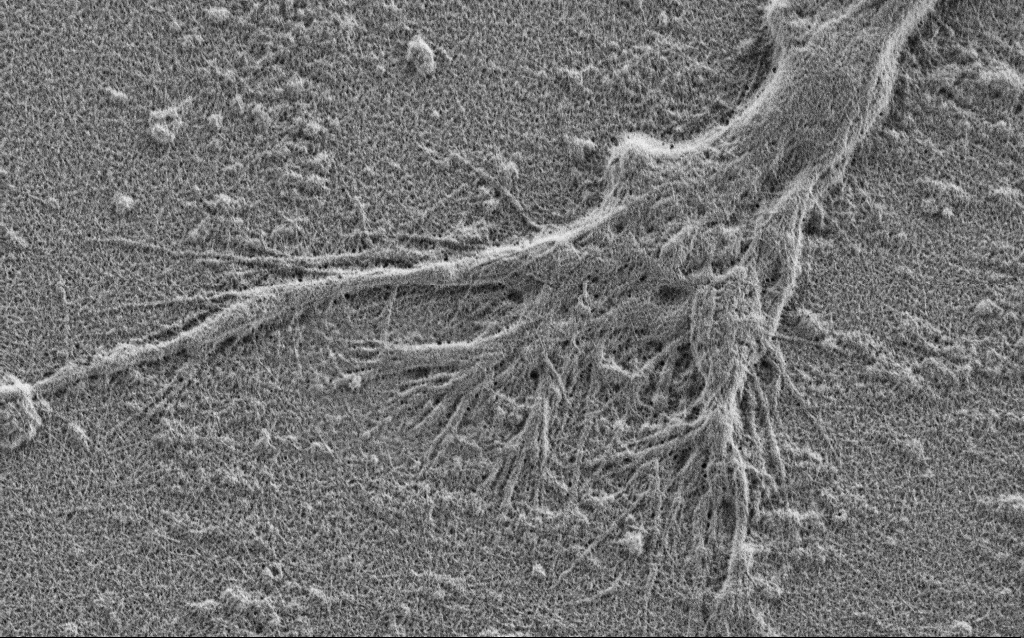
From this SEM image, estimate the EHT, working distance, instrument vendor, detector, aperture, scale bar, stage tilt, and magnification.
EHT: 1 kV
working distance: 4 mm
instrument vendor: Zeiss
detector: SE2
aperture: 30 µm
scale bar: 2000 nm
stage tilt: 0°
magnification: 10 K X